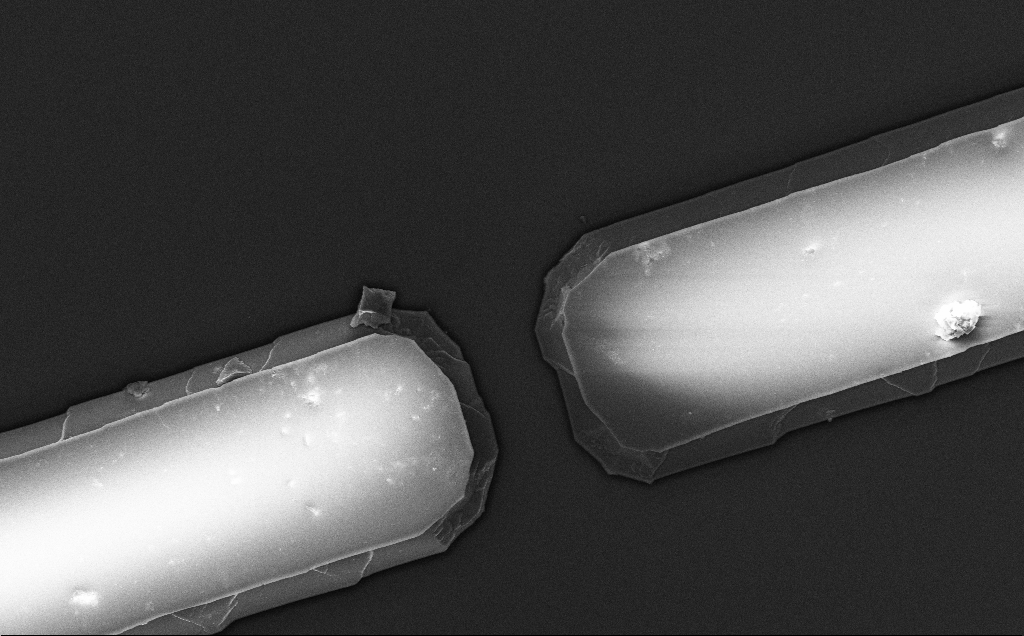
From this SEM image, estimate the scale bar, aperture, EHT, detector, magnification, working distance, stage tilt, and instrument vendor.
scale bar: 10000 nm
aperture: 30 µm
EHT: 5 kV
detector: InLens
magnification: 5.6 K X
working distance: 10 mm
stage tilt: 0°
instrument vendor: Zeiss